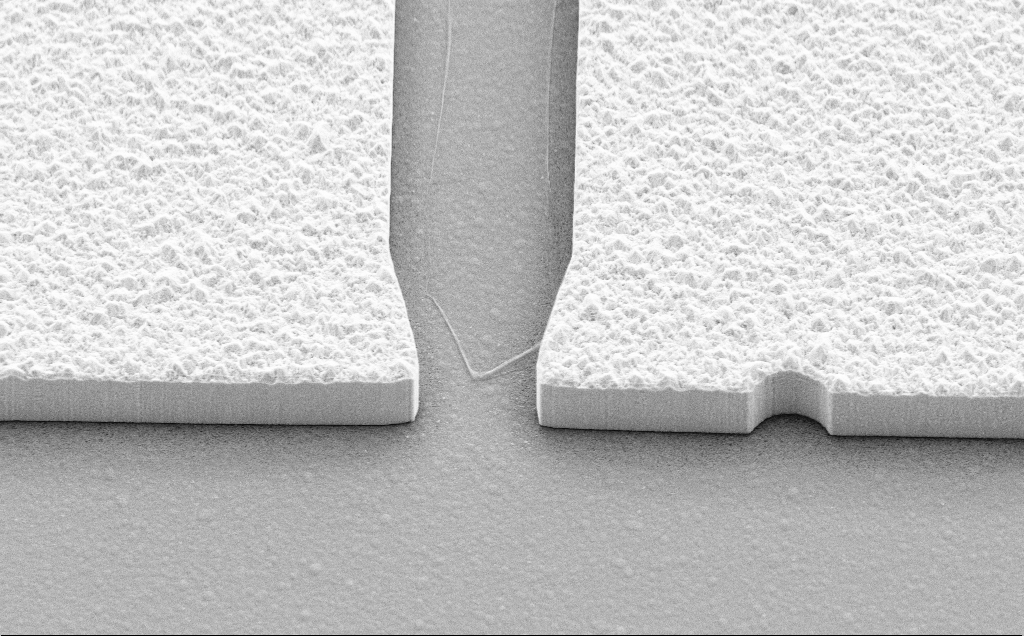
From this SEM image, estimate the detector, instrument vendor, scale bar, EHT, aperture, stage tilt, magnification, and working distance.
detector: SE2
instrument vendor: Zeiss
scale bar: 10000 nm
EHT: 10 kV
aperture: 30 µm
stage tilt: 45°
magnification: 6.62 K X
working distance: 8 mm